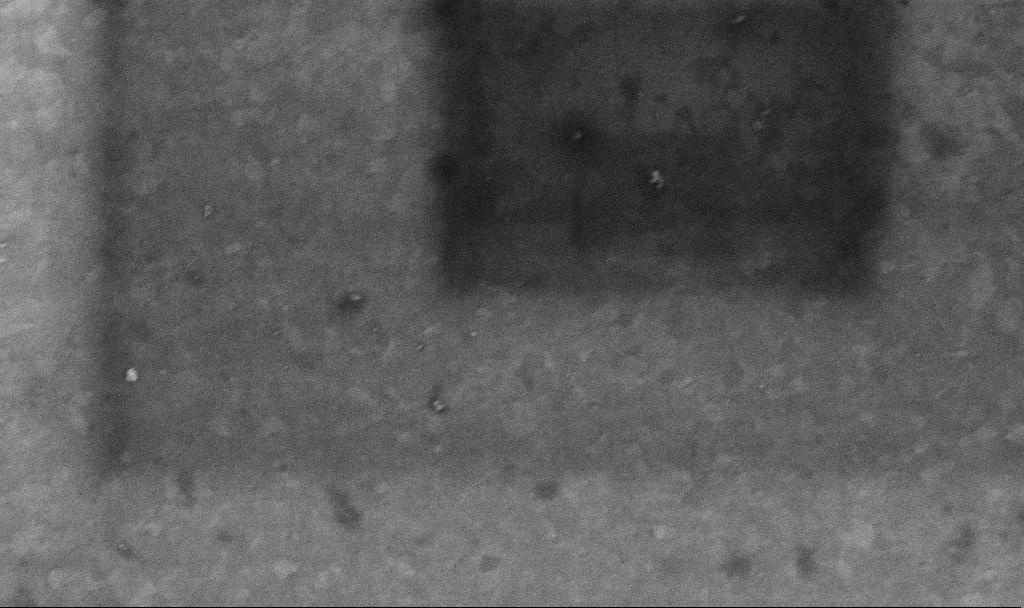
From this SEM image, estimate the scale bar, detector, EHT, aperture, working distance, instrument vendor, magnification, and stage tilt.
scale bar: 200 nm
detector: InLens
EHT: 10 kV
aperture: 30 µm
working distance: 3.4 mm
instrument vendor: Zeiss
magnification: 99.94 K X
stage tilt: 0°